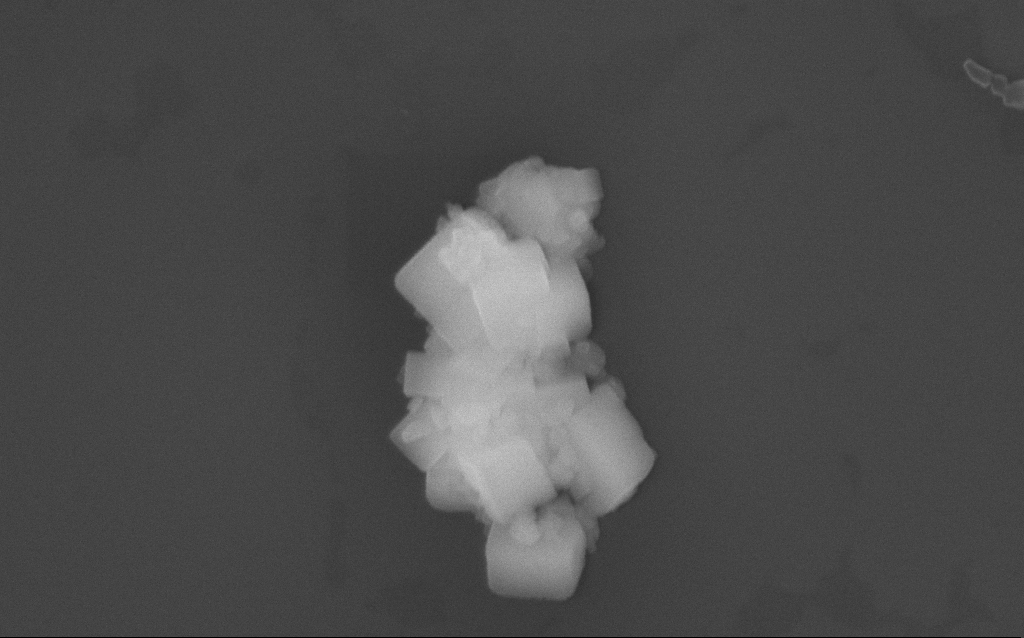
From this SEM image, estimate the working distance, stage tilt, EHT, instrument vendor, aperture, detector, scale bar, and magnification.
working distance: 3 mm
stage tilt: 0°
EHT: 10 kV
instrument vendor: Zeiss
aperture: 30 µm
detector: InLens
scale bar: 1000 nm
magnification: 70.2 K X